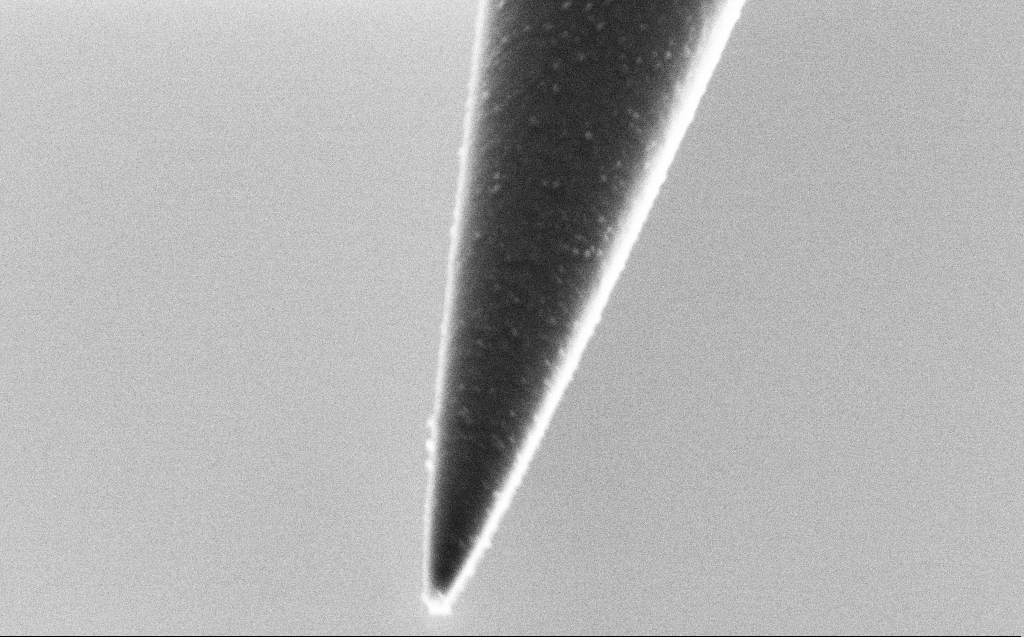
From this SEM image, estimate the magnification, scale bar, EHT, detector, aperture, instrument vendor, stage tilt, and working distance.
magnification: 85.25 K X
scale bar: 200 nm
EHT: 2 kV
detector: SE2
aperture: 30 µm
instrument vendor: Zeiss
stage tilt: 45°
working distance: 5 mm